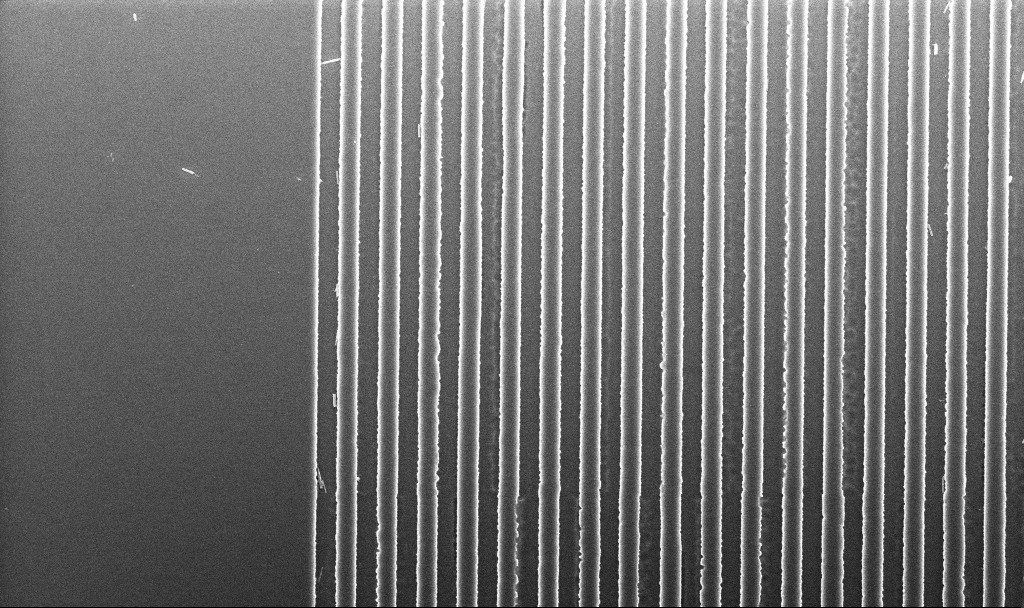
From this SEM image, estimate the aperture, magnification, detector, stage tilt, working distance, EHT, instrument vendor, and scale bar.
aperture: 30 µm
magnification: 22.82 K X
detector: InLens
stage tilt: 0°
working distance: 3 mm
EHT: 3 kV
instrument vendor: Zeiss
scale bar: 2000 nm